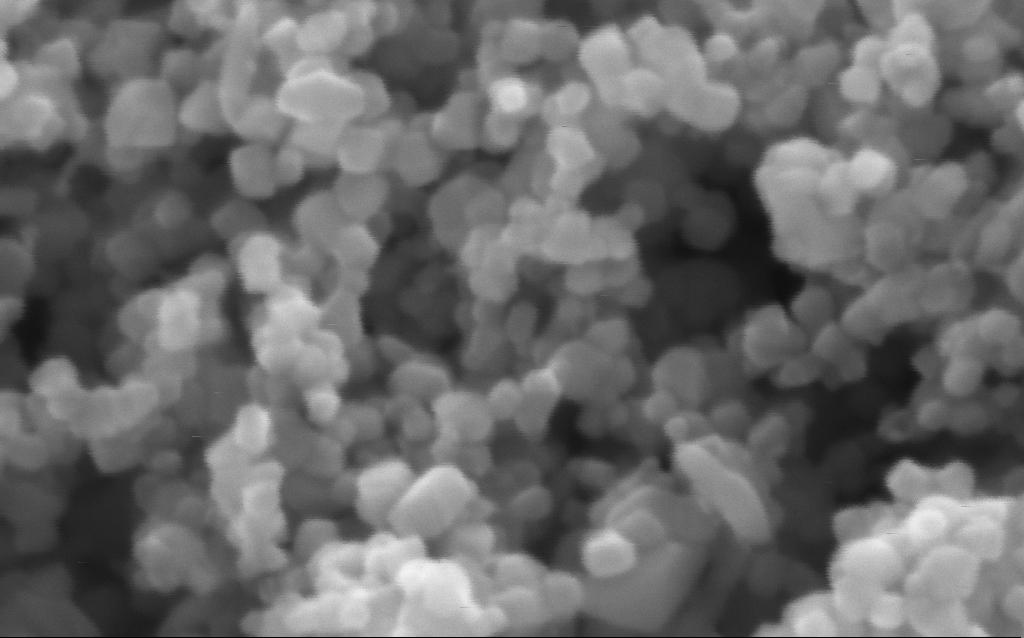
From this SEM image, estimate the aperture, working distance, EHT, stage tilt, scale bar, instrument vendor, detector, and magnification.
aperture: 30 µm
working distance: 4.4 mm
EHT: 5 kV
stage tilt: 0°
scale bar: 100 nm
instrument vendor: Zeiss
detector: InLens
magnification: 716 K X